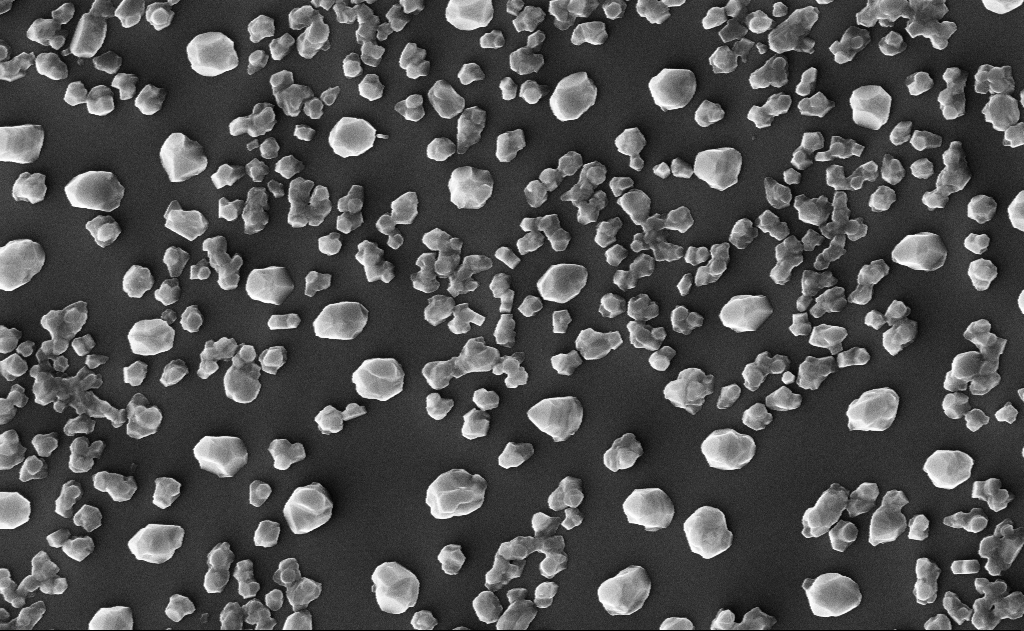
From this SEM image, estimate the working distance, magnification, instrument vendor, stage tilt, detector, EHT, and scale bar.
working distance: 16 mm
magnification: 40 K X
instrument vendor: Zeiss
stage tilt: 0°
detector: InLens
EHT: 10 kV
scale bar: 1000 nm